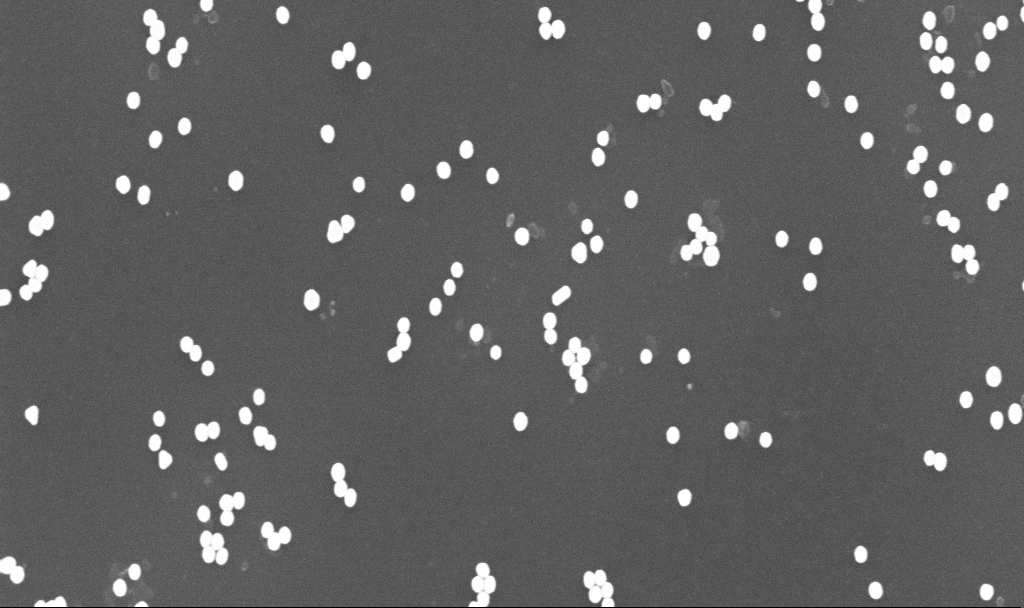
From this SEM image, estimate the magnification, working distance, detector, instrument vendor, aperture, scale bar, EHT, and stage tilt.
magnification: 70 K X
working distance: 3.4 mm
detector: InLens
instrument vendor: Zeiss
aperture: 30 µm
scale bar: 1000 nm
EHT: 10 kV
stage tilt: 0°